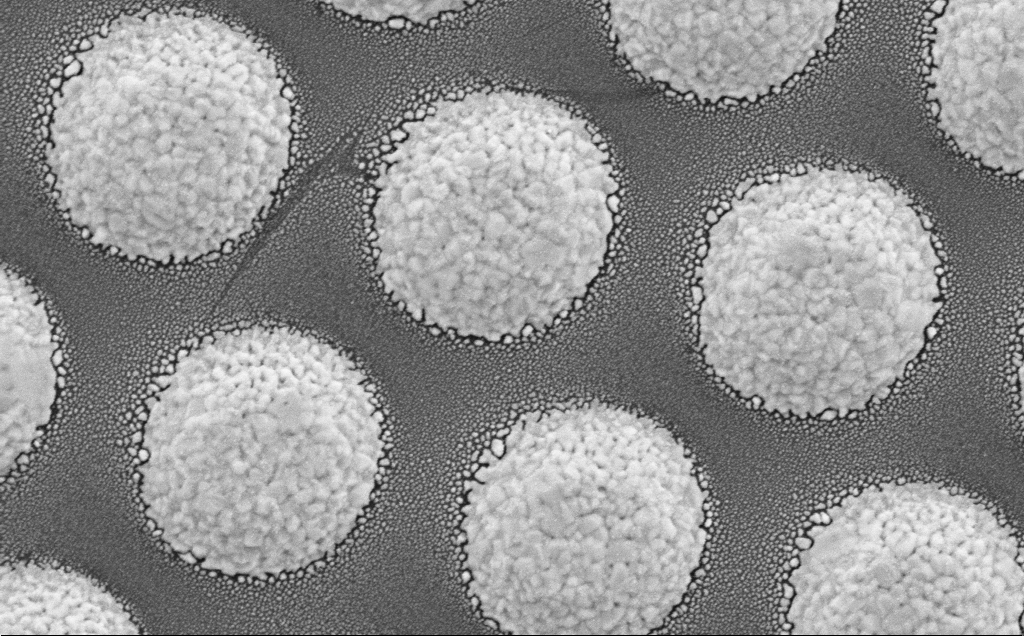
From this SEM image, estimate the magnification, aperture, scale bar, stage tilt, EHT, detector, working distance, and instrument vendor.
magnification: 40.48 K X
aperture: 30 µm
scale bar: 1000 nm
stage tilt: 0°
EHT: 5 kV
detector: SE2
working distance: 15 mm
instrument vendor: Zeiss